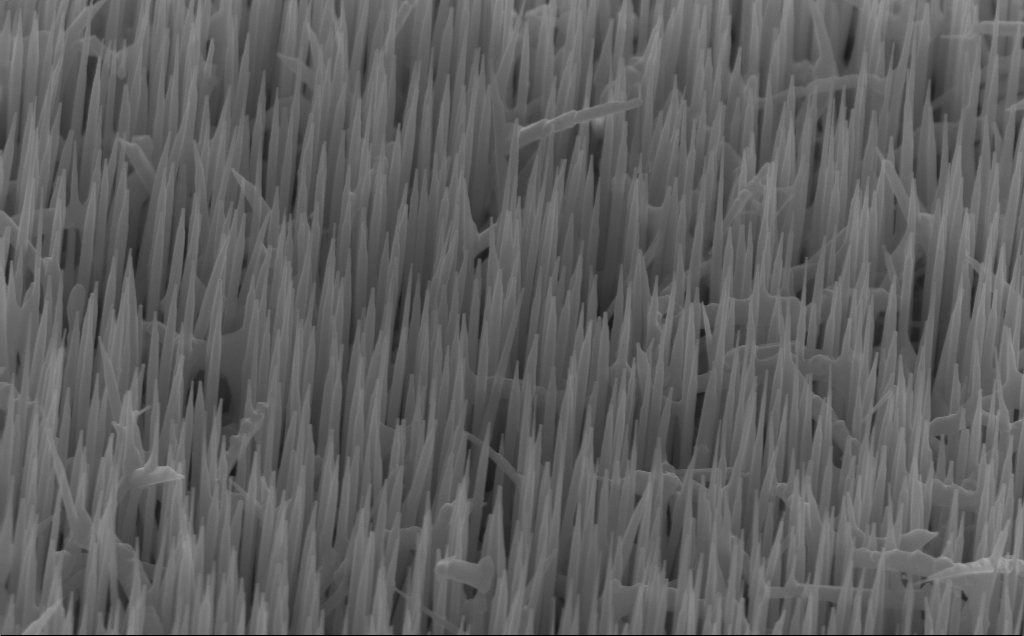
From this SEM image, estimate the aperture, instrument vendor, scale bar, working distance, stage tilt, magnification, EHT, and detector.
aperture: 30 µm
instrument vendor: Zeiss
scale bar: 1000 nm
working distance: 5 mm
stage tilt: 45°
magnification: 40 K X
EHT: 12 kV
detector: InLens